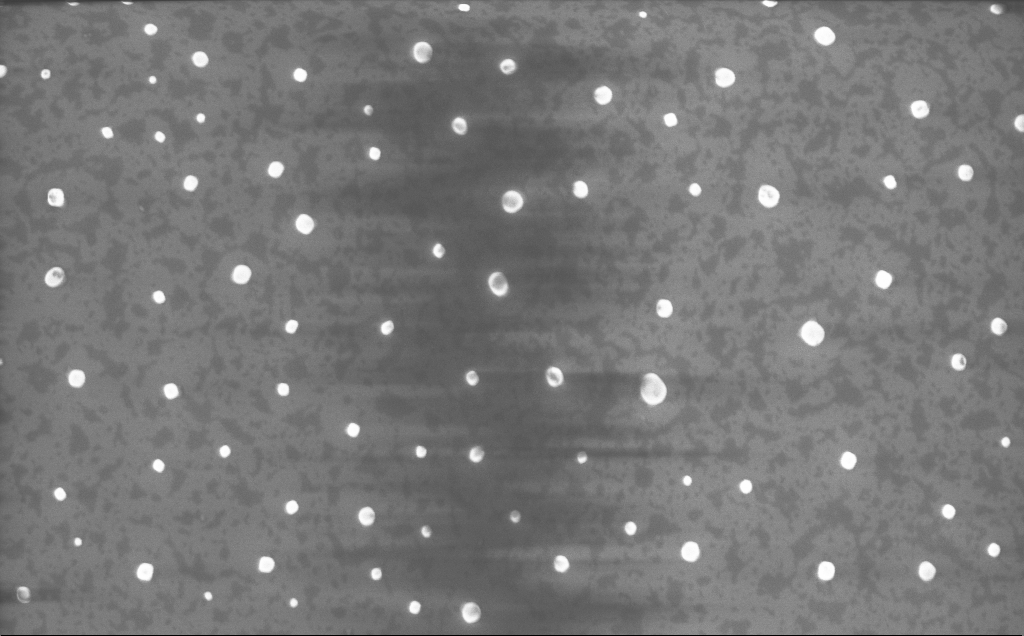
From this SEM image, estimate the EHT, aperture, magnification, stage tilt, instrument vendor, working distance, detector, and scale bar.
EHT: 10 kV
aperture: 30 µm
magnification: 31.17 K X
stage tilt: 0°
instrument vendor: Zeiss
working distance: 4 mm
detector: InLens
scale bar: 2000 nm